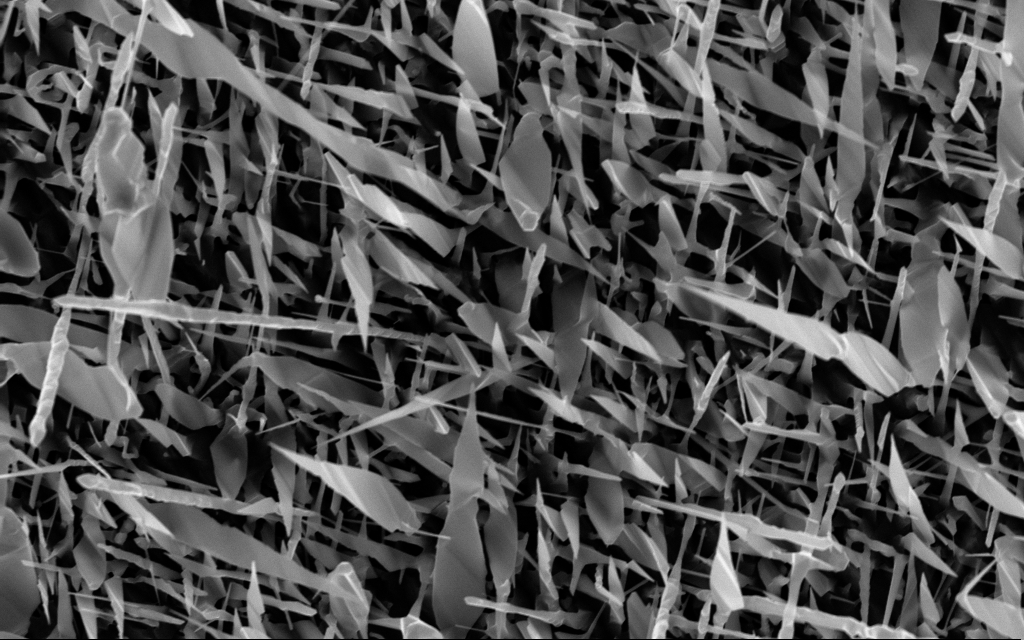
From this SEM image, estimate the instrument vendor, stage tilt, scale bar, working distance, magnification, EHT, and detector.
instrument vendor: Zeiss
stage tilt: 0°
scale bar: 2000 nm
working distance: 6 mm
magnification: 20 K X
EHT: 10 kV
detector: InLens